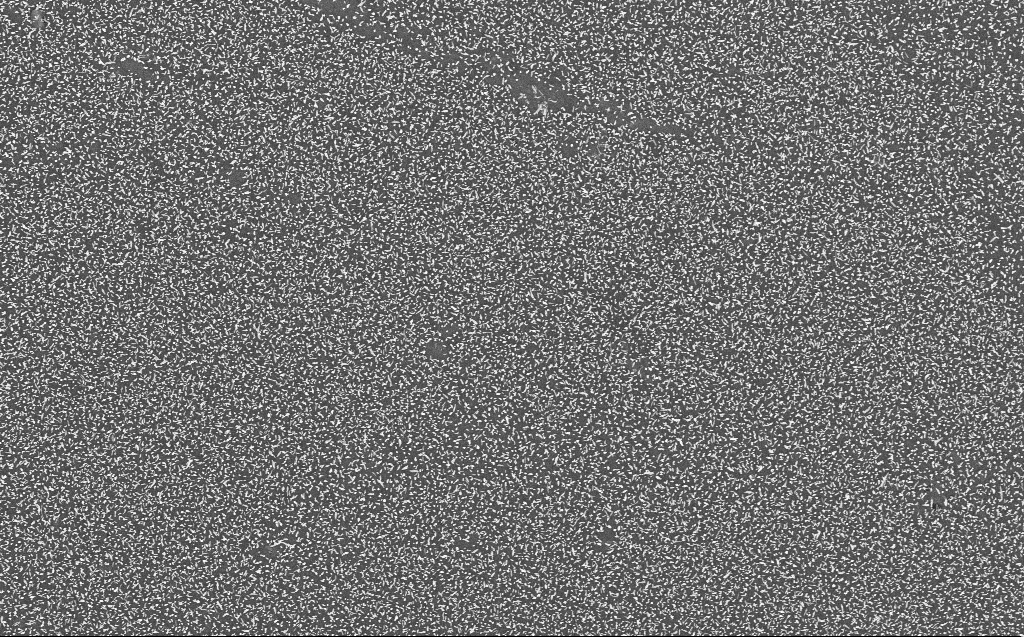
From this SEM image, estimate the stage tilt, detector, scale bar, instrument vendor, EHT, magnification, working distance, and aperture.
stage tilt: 0°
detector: InLens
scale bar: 10000 nm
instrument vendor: Zeiss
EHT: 10 kV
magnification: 5 K X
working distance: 3 mm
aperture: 30 µm